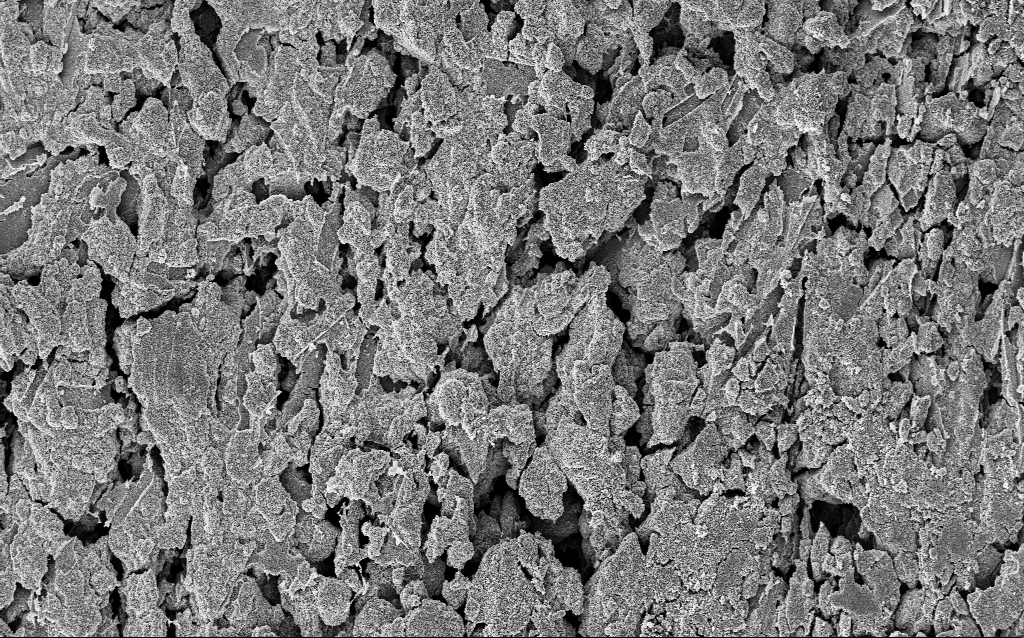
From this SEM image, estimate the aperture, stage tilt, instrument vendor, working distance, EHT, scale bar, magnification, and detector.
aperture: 30 µm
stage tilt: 0°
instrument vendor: Zeiss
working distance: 9.6 mm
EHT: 5 kV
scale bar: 20000 nm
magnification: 1.23 K X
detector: InLens